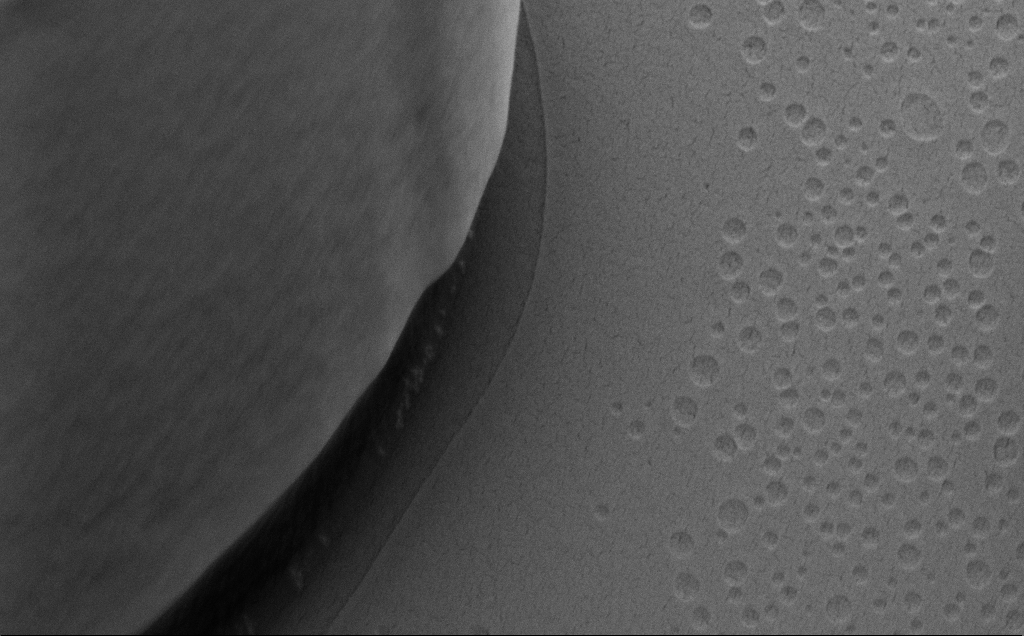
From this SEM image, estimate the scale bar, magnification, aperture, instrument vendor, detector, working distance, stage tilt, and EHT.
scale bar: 1000 nm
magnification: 50 K X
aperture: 30 µm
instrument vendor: Zeiss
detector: SE2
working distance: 8 mm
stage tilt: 40°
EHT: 5 kV